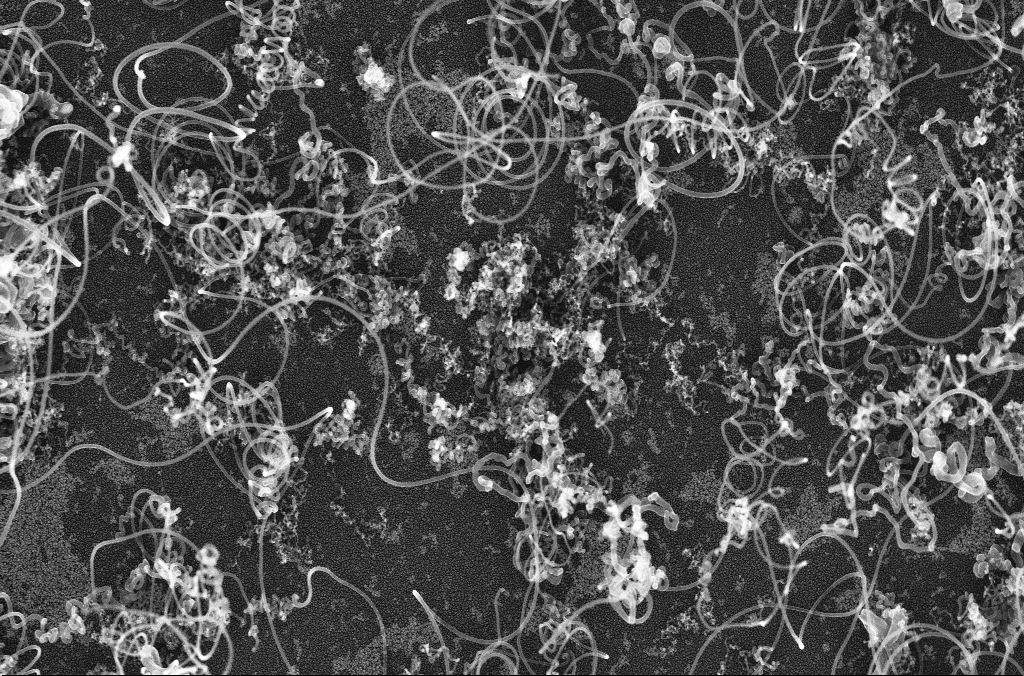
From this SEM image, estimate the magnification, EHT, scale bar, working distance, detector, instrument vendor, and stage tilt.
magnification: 10 K X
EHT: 20 kV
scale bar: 2000 nm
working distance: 4.2 mm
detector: InLens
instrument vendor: Zeiss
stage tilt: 0°